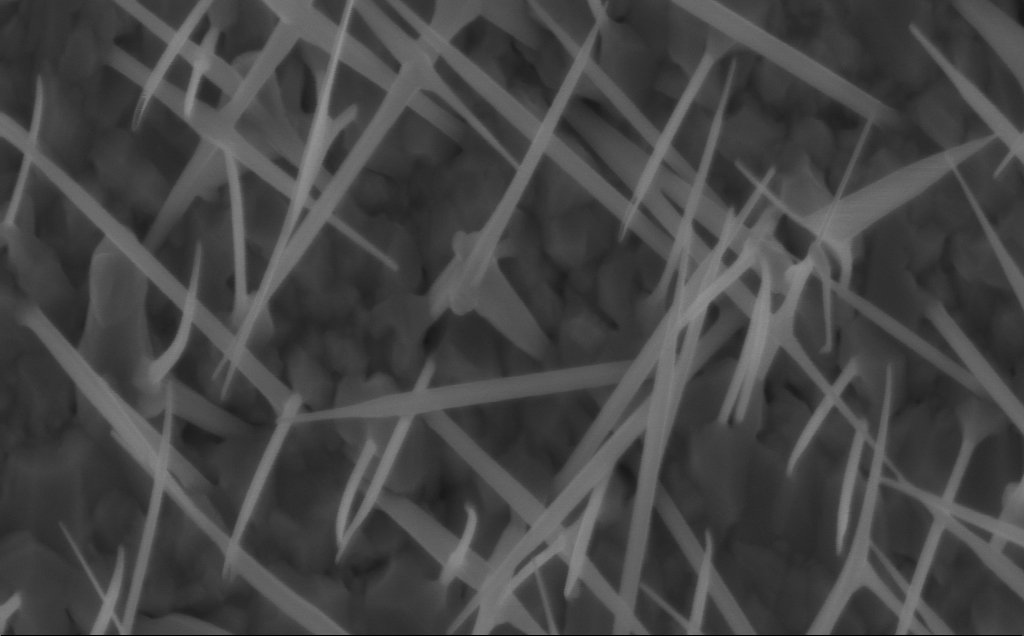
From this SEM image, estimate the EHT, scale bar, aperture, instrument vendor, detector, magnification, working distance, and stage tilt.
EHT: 5 kV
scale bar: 200 nm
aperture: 30 µm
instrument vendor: Zeiss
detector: InLens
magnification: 80 K X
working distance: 5 mm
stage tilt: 0°